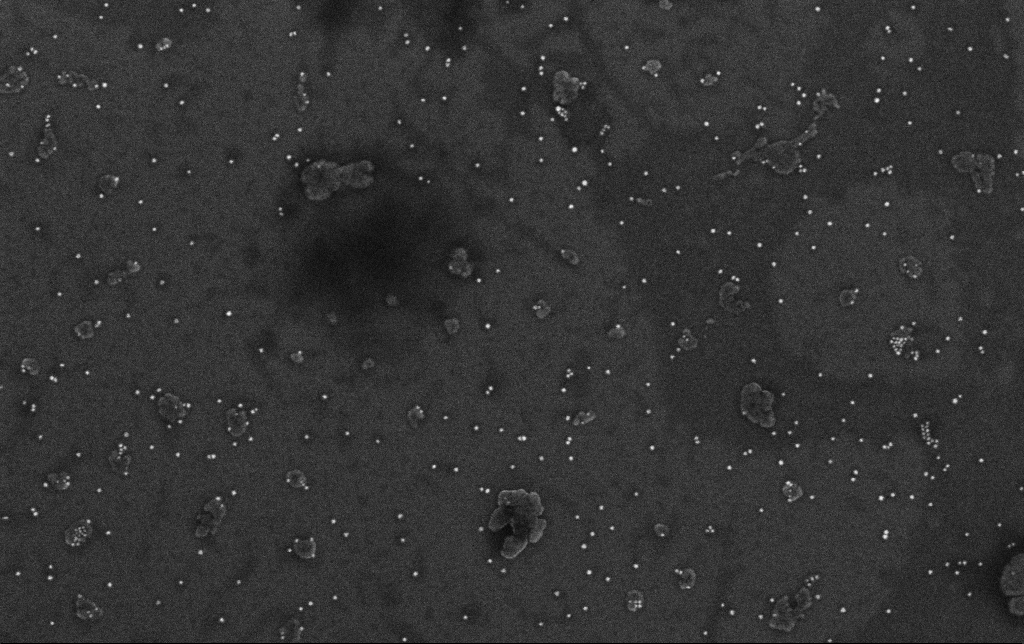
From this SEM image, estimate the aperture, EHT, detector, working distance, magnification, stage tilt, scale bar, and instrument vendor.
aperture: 30 µm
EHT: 10 kV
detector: InLens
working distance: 3.2 mm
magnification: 100 K X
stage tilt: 0°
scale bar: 200 nm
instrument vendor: Zeiss